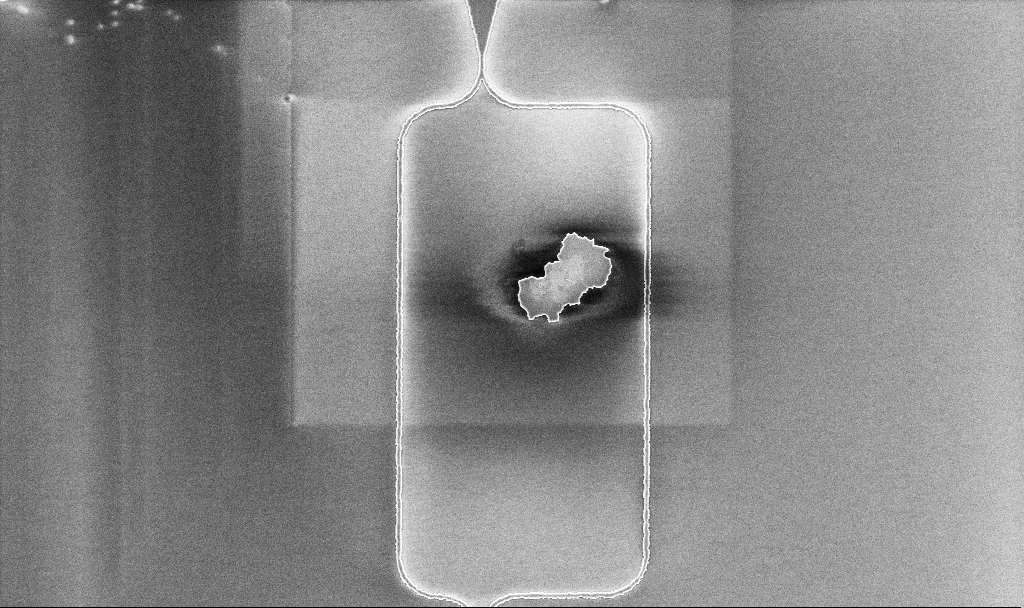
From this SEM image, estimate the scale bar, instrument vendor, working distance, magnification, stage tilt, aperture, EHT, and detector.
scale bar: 10000 nm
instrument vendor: Zeiss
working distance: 10.1 mm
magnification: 3.16 K X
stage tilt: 0°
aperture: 30 µm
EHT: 5 kV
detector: InLens